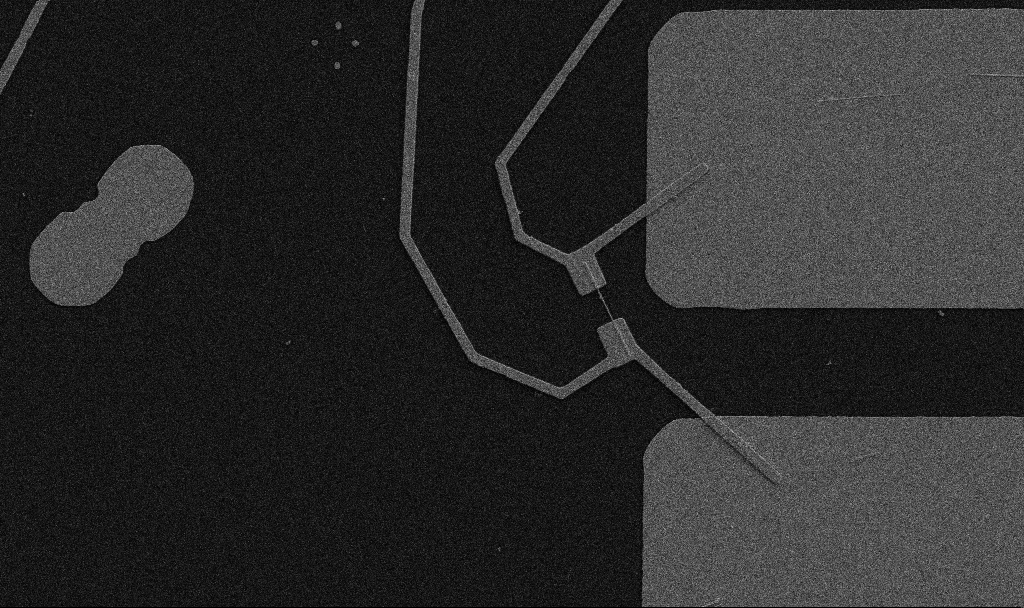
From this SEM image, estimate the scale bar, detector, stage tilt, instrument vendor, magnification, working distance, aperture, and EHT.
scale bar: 10000 nm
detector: SE2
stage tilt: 0°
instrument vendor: Zeiss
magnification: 5 K X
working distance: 10.7 mm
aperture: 30 µm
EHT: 5 kV